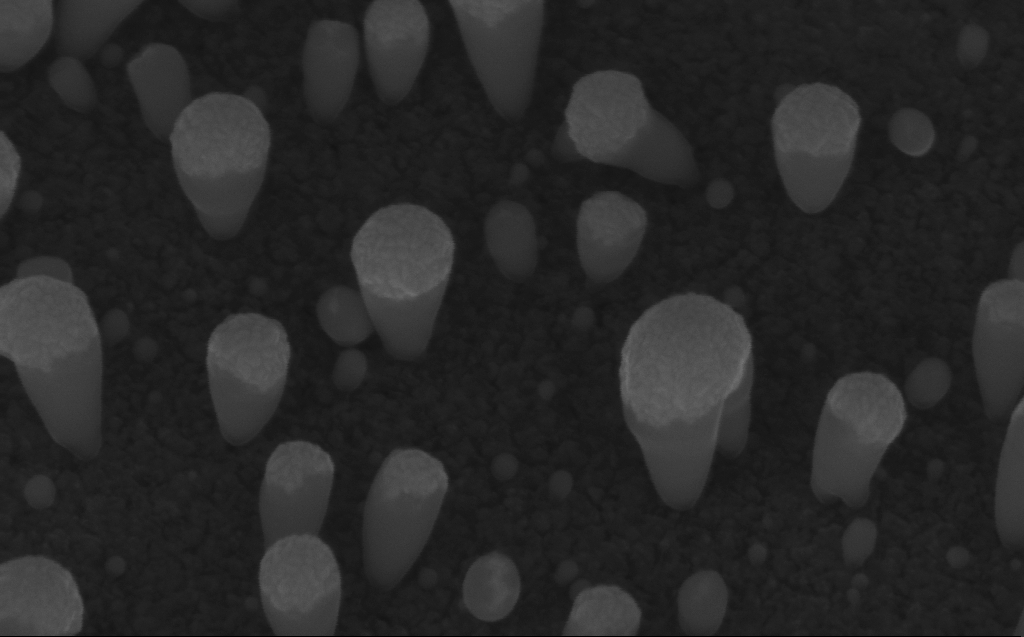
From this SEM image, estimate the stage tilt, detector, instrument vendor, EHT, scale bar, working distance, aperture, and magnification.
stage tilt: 45°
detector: InLens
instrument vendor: Zeiss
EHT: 10 kV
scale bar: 100 nm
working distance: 7 mm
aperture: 30 µm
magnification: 200 K X